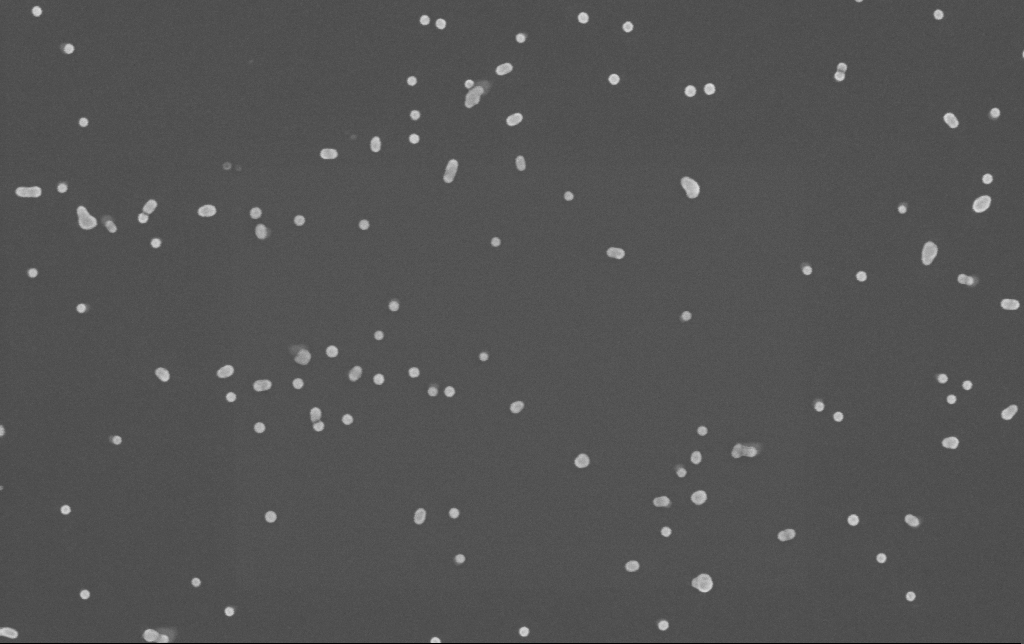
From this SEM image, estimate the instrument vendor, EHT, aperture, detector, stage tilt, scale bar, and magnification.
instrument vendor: Zeiss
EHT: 10 kV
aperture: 30 µm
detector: InLens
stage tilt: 0°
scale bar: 200 nm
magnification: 150 K X